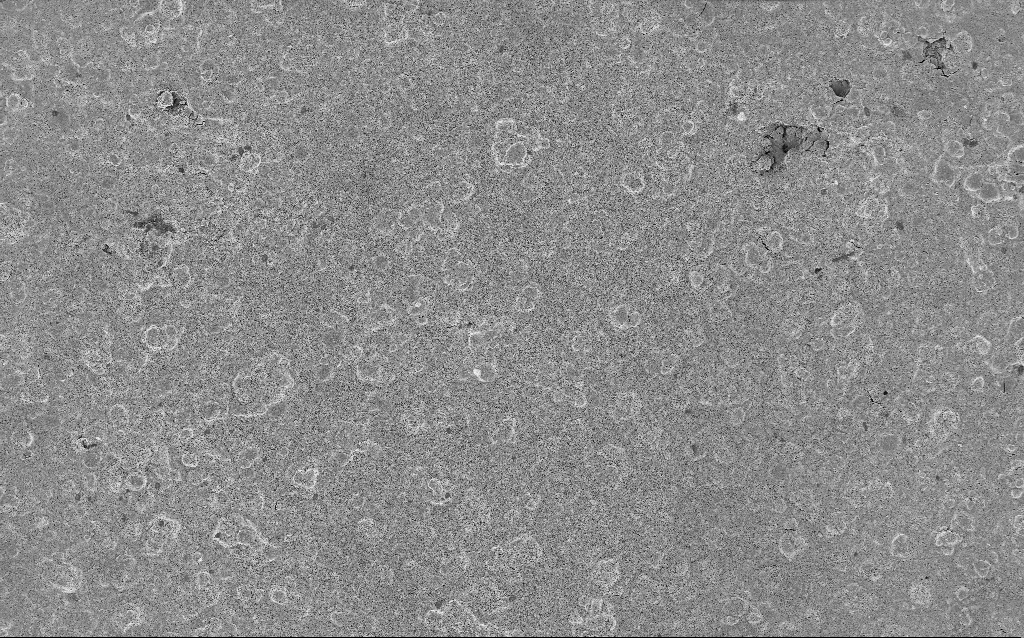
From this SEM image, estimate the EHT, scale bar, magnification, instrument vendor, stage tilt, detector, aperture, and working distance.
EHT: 3 kV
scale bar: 20000 nm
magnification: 0.77 K X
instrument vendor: Zeiss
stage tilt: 0°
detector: InLens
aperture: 30 µm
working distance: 7.5 mm